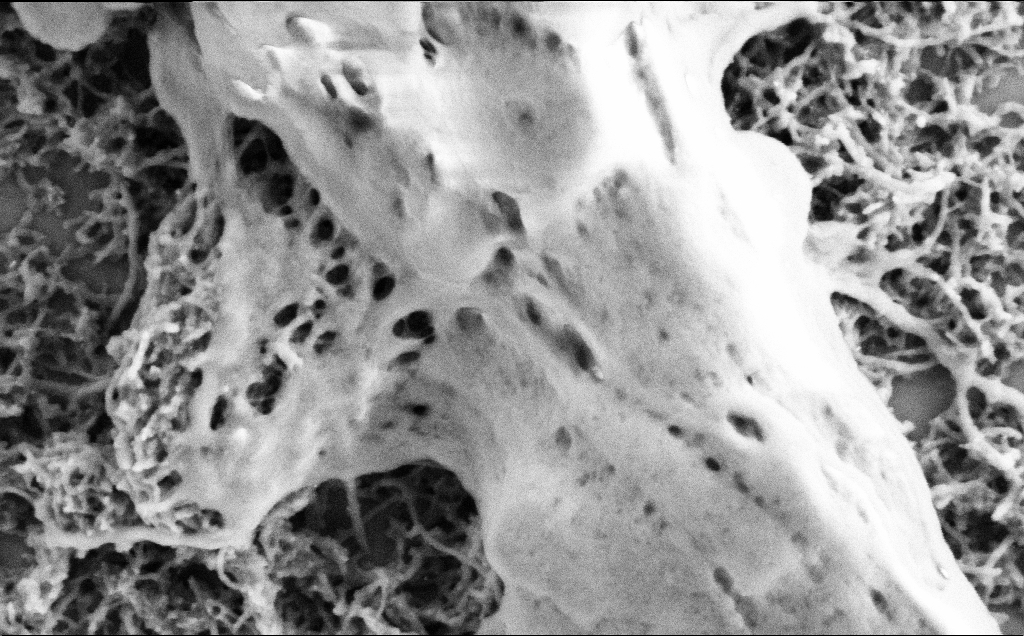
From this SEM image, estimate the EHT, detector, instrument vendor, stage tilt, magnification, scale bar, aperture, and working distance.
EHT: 2 kV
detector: SE2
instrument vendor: Zeiss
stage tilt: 0°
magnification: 75 K X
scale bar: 200 nm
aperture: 30 µm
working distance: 7.1 mm